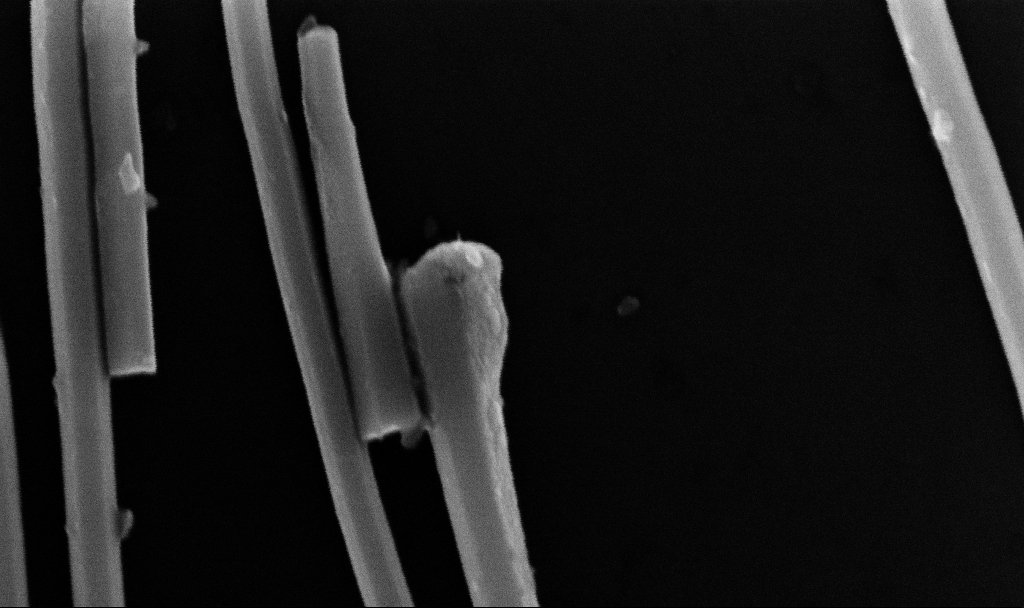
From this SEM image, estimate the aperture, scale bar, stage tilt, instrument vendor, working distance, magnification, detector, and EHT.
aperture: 30 µm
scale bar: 200 nm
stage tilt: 0°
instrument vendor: Zeiss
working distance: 8.7 mm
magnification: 168.08 K X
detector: InLens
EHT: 10 kV